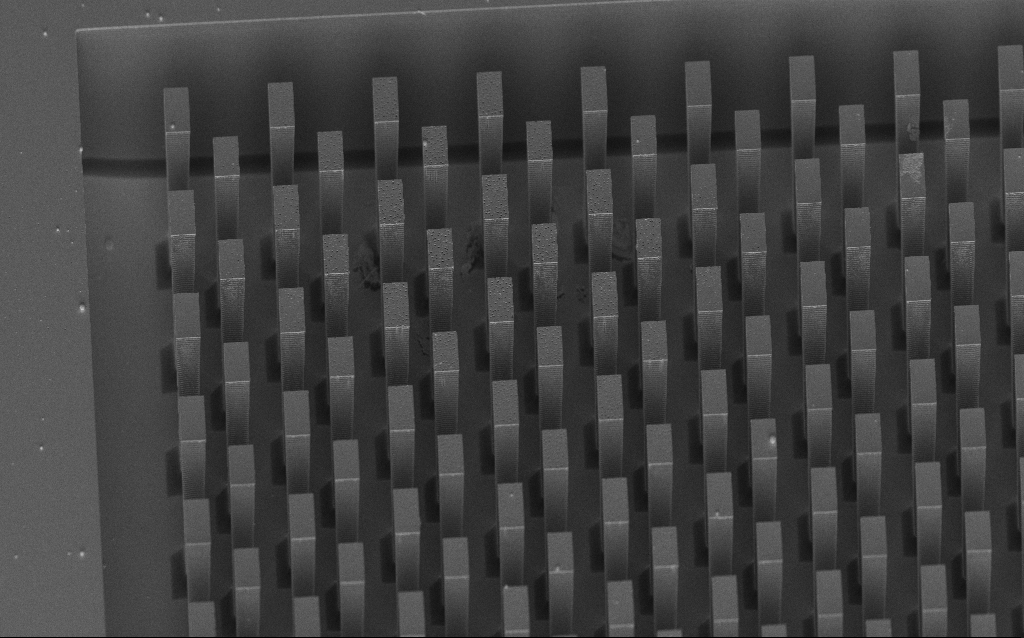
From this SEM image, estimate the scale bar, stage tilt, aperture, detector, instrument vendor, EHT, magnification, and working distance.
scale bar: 10000 nm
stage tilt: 45°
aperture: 30 µm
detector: InLens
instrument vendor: Zeiss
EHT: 5 kV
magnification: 1.91 K X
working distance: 8 mm